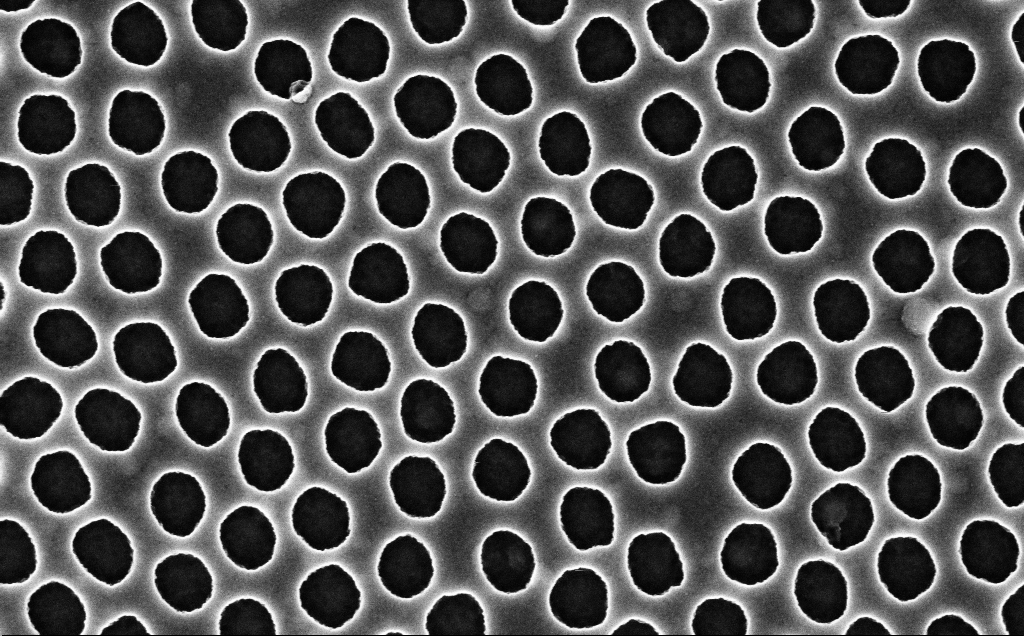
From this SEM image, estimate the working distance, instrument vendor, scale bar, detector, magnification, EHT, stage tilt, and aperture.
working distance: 2.5 mm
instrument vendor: Zeiss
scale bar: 200 nm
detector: InLens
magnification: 90 K X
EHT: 3 kV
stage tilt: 0°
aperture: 30 µm